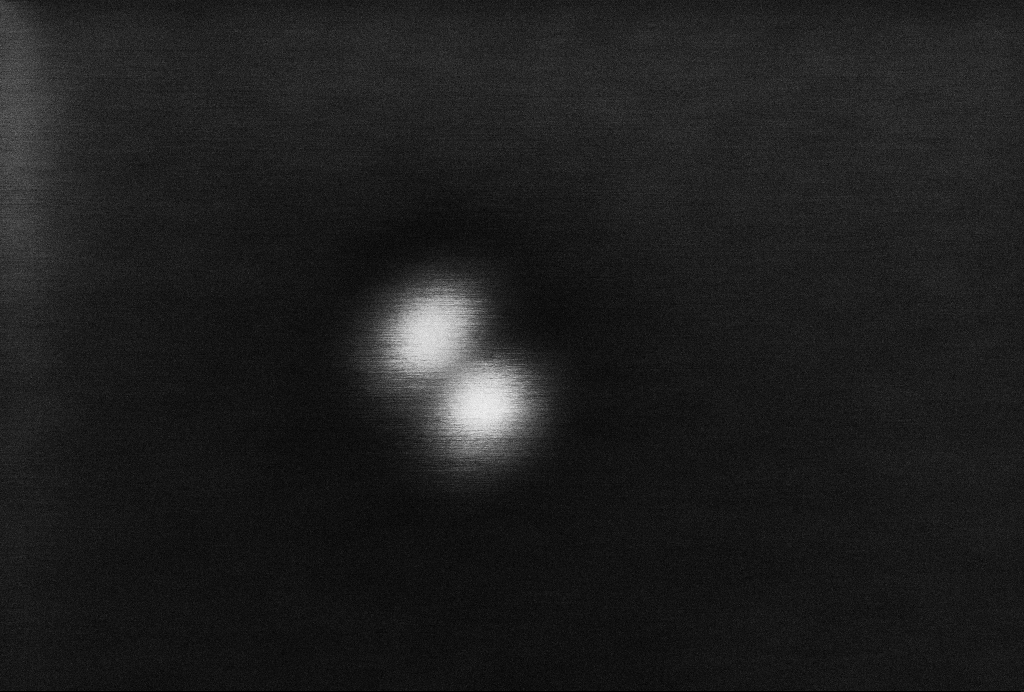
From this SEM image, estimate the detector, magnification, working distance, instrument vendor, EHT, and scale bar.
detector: InLens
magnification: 1537.16 K X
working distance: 3.3 mm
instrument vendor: Zeiss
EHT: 2 kV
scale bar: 20 nm